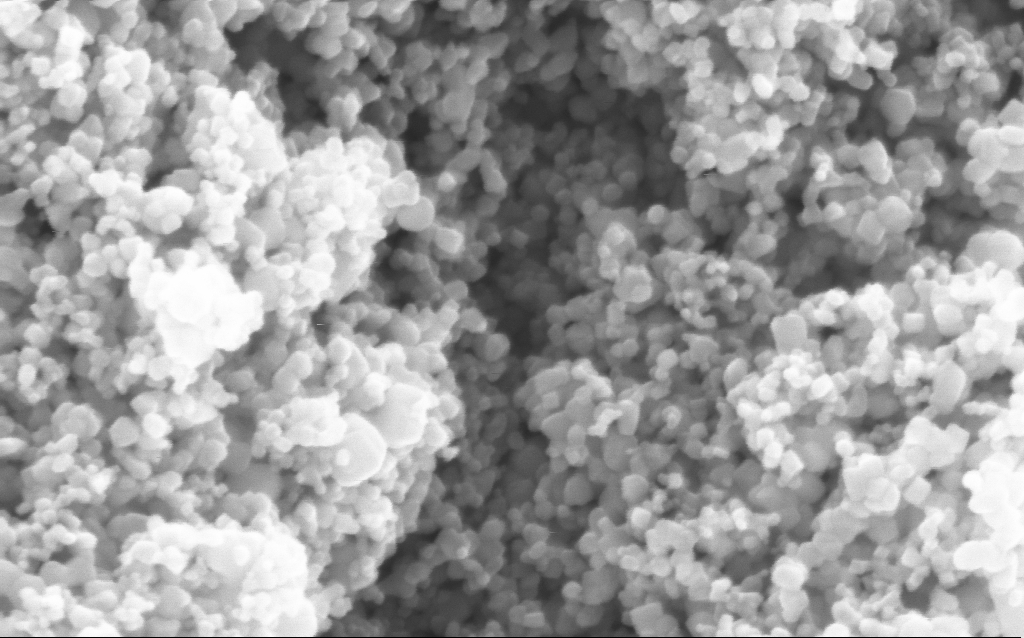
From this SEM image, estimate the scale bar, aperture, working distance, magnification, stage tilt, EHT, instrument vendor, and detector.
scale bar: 200 nm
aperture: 30 µm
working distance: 4.4 mm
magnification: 294.51 K X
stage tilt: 0°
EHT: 5 kV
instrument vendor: Zeiss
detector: InLens